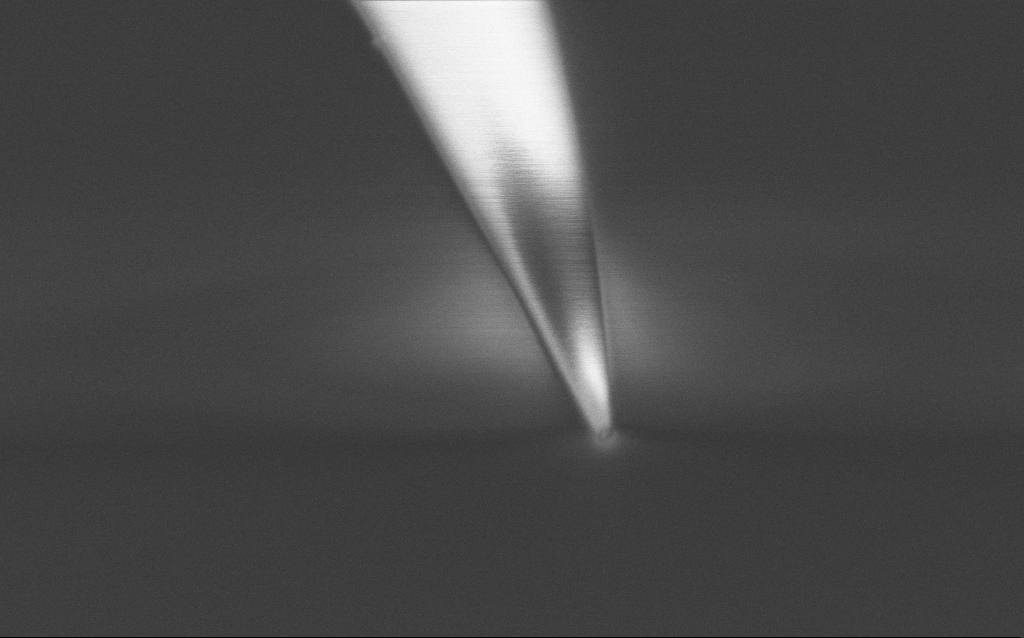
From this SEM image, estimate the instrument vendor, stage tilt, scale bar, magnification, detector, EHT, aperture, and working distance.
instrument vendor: Zeiss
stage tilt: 45°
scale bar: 1000 nm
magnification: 50 K X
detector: InLens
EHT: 1 kV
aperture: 30 µm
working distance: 7 mm